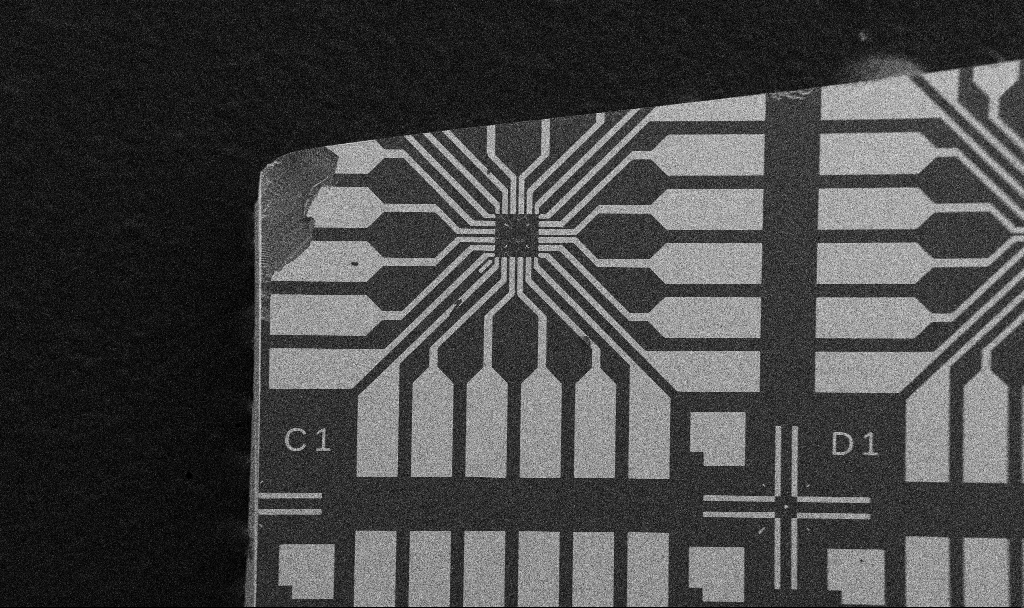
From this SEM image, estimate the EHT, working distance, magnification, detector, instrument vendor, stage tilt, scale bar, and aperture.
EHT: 5 kV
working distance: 10.7 mm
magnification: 0.1 K X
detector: SE2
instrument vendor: Zeiss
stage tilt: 0°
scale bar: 200000 nm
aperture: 30 µm